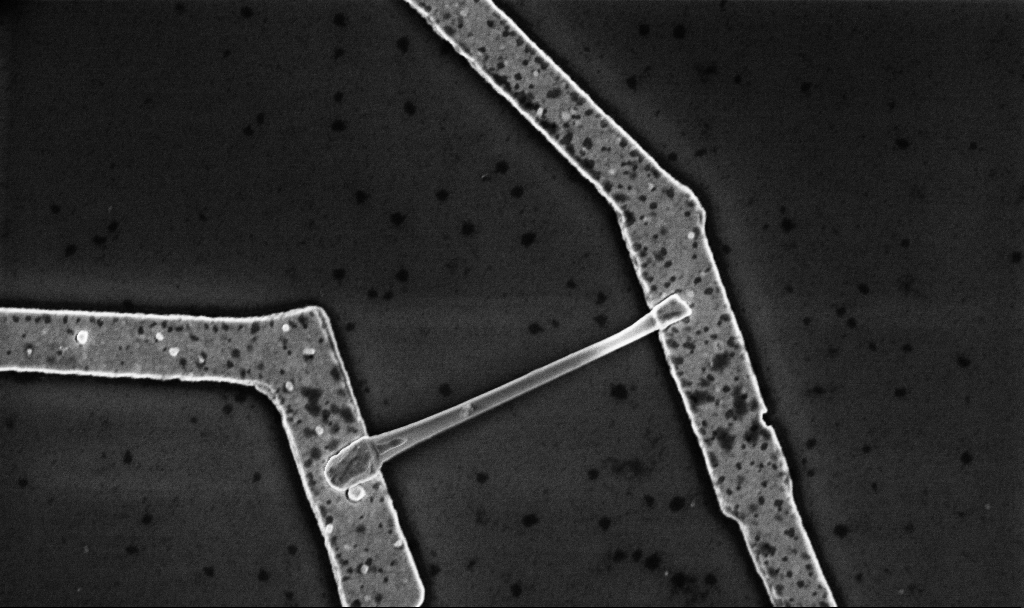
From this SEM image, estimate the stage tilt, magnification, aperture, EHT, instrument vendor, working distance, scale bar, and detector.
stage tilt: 0°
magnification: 30 K X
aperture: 30 µm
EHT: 5 kV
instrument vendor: Zeiss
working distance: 8.7 mm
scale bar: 1000 nm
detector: InLens